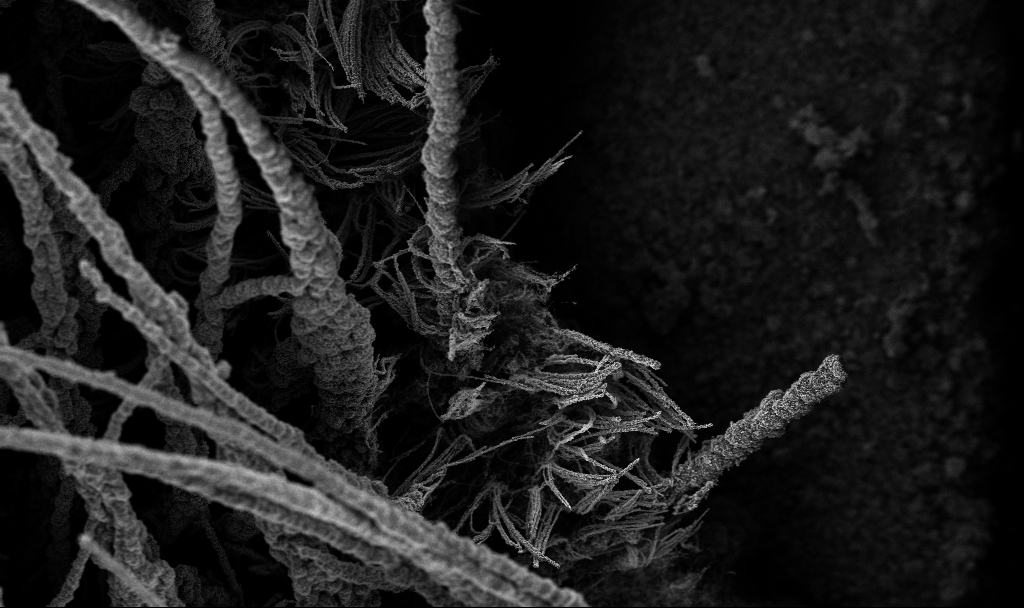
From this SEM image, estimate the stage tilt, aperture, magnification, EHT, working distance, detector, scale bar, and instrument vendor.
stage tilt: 0°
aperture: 30 µm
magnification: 0.25 K X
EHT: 3 kV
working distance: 3.4 mm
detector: InLens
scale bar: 100000 nm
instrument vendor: Zeiss